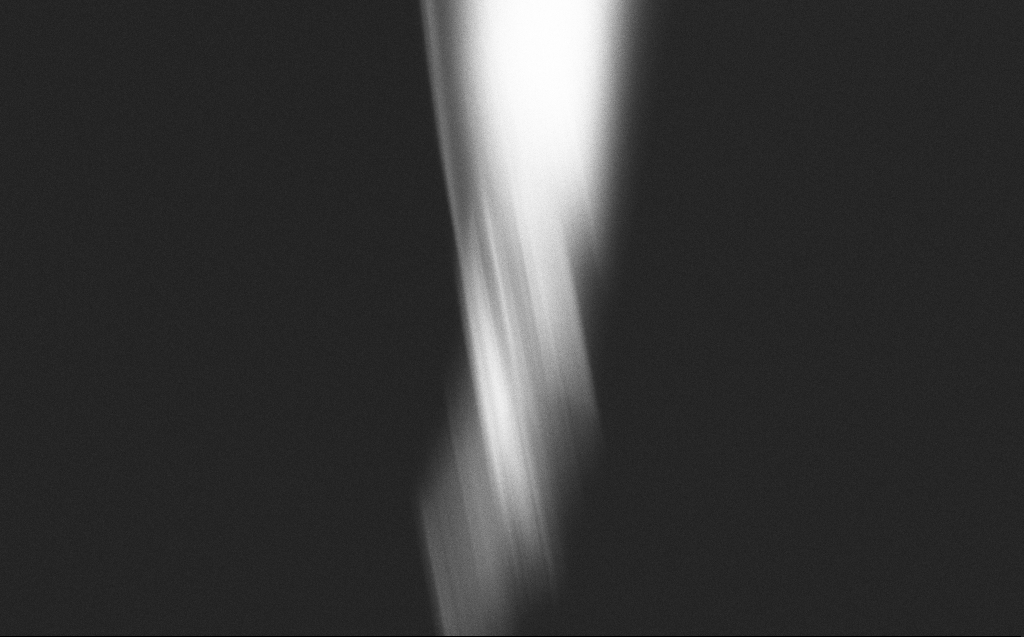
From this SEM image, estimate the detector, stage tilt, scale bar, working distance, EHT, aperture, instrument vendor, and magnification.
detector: InLens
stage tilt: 45°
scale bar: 200 nm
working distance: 5 mm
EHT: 5 kV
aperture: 20 µm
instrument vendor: Zeiss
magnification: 78.58 K X